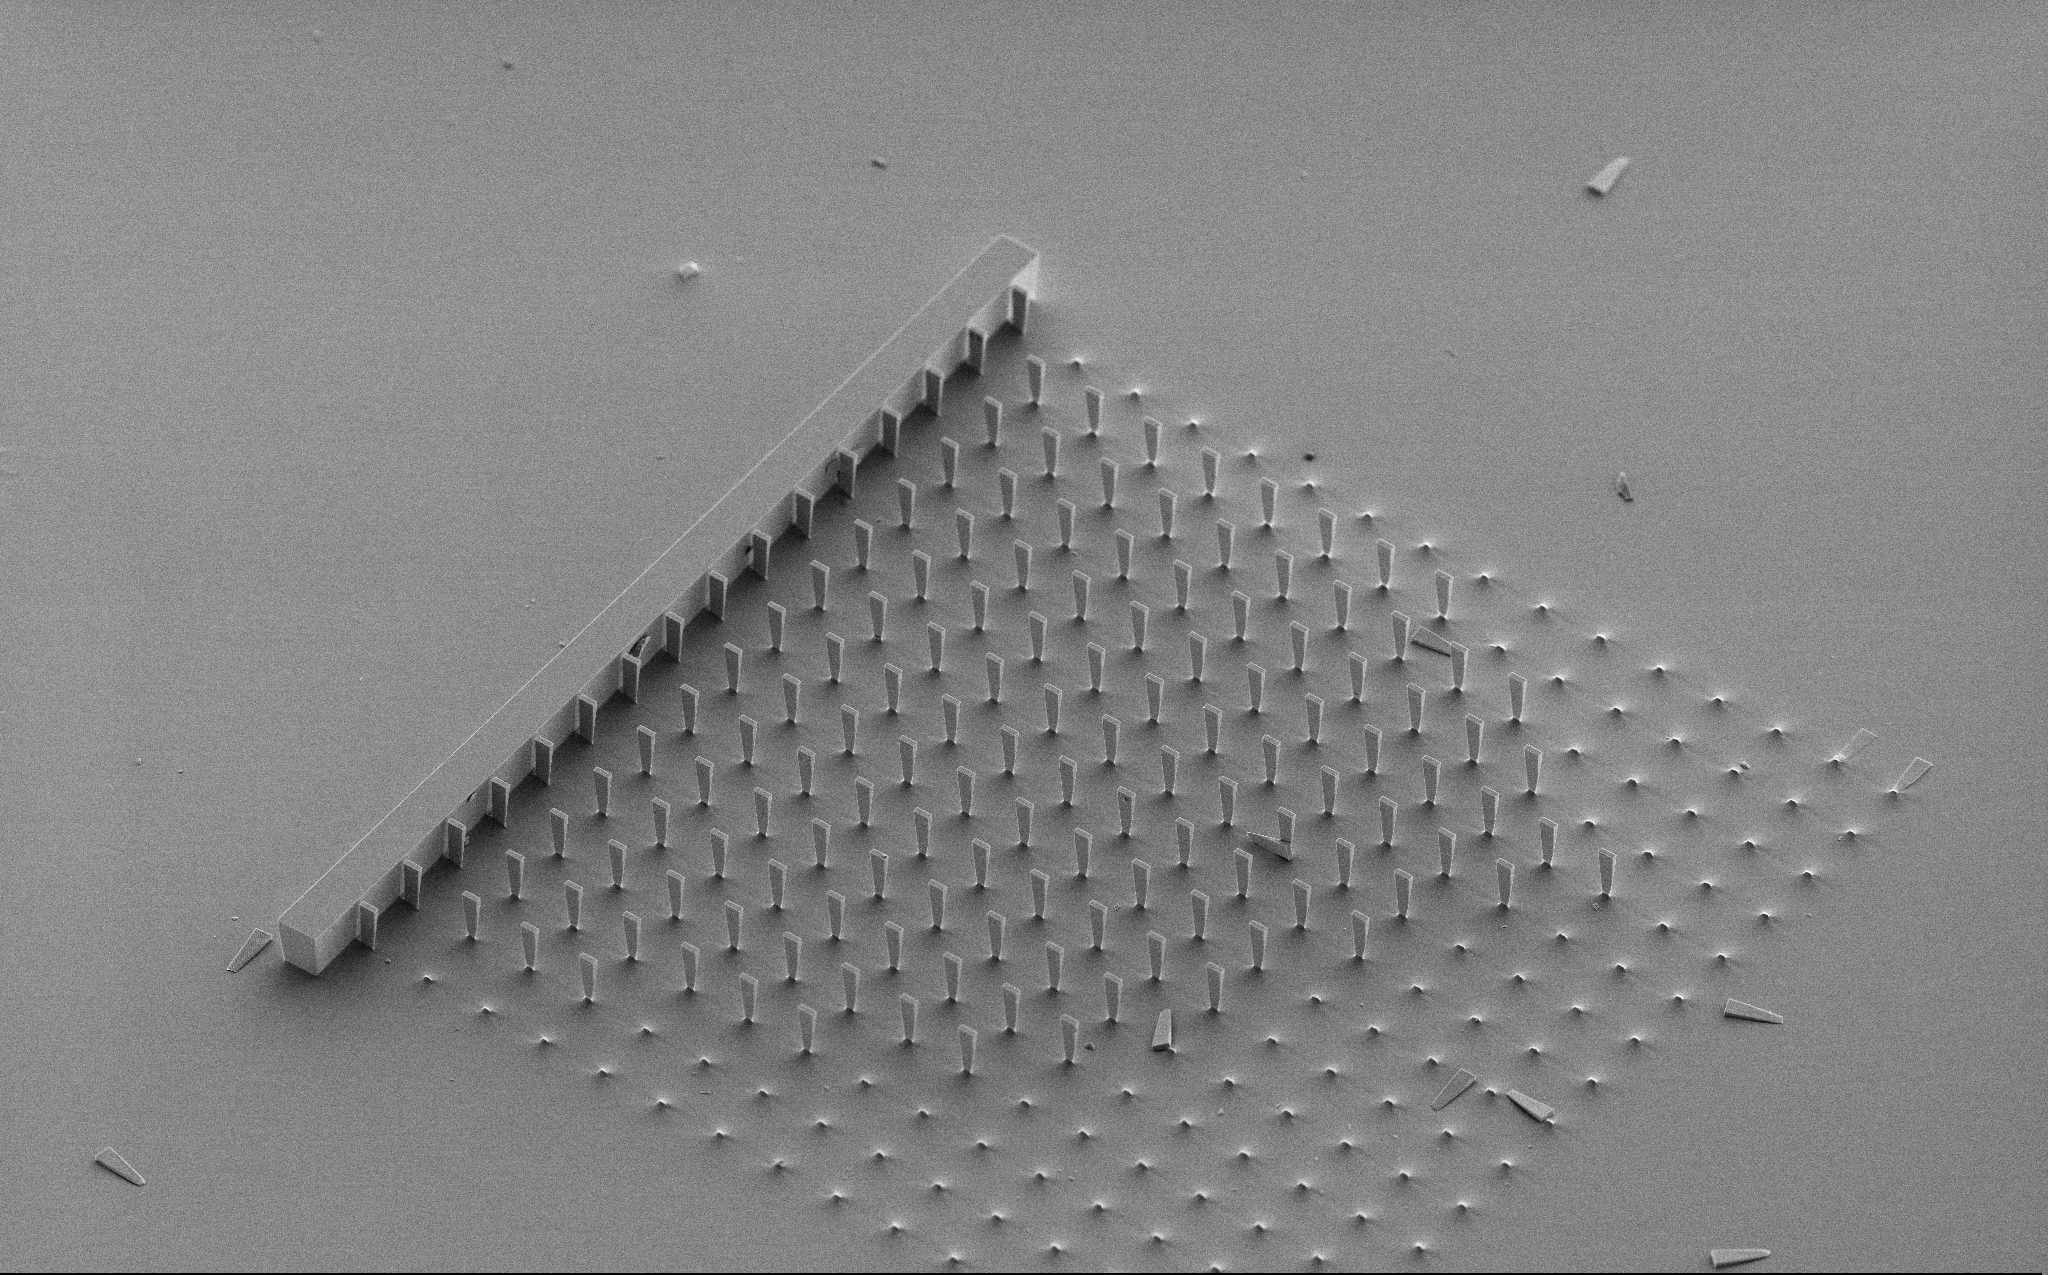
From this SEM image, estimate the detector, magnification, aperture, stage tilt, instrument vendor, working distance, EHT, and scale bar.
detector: SE2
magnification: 0.447 K X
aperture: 30 µm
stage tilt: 45°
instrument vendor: Zeiss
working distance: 10 mm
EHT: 5 kV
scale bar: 100000 nm